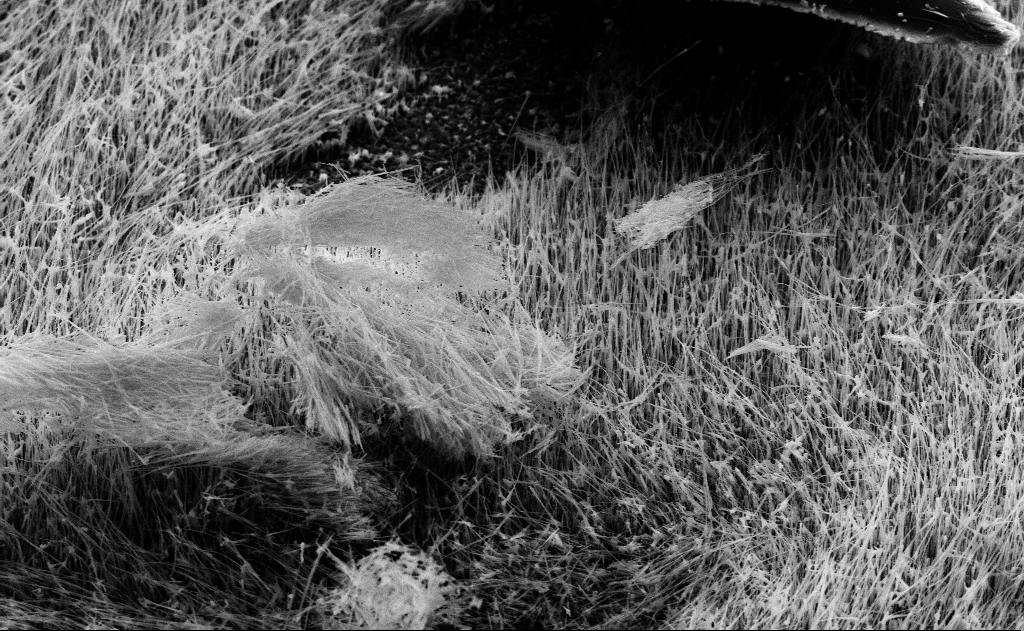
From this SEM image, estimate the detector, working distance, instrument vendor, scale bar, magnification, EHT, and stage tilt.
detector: SE2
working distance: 15 mm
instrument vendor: Zeiss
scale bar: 2000 nm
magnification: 10 K X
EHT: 10 kV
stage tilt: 45°